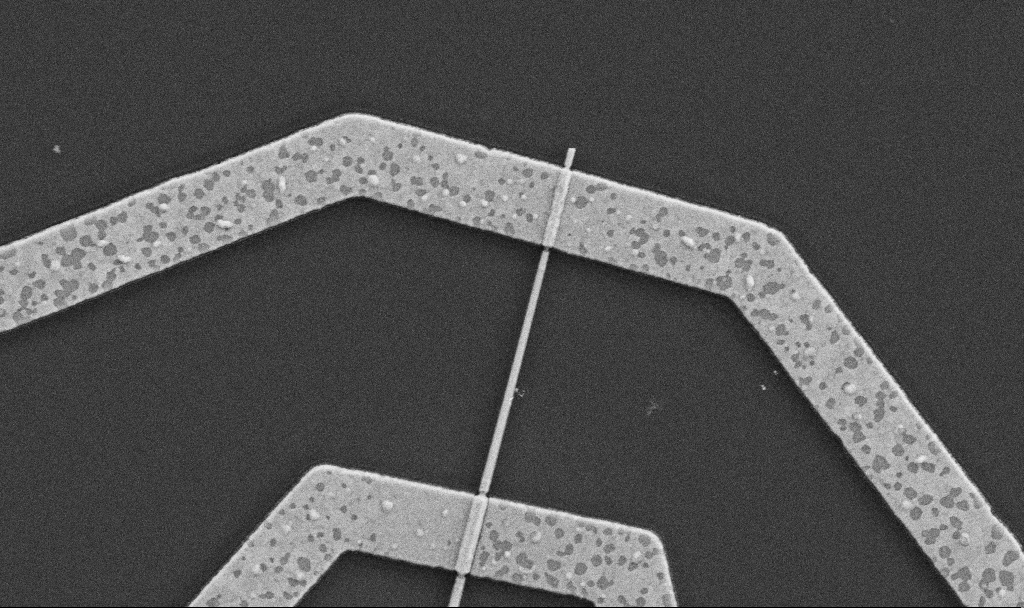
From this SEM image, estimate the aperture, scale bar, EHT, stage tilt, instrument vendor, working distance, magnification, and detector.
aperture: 30 µm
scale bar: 1000 nm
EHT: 5 kV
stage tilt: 0°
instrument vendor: Zeiss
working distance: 8.7 mm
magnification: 30 K X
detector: SE2